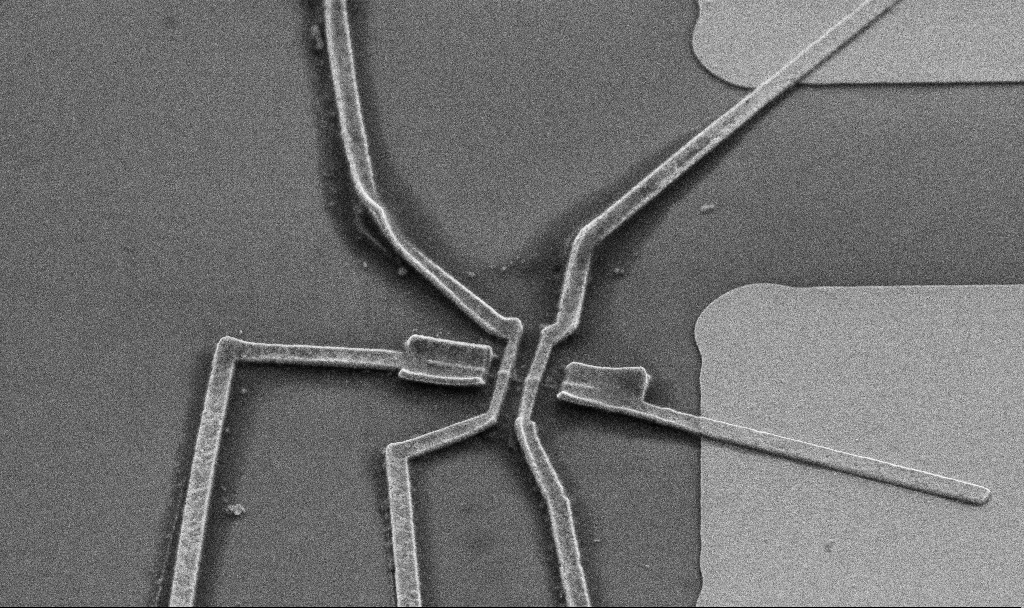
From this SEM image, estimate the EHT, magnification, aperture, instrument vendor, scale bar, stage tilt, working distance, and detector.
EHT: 5 kV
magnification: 10 K X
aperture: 30 µm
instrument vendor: Zeiss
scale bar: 2000 nm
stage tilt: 45°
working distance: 12.4 mm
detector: SE2